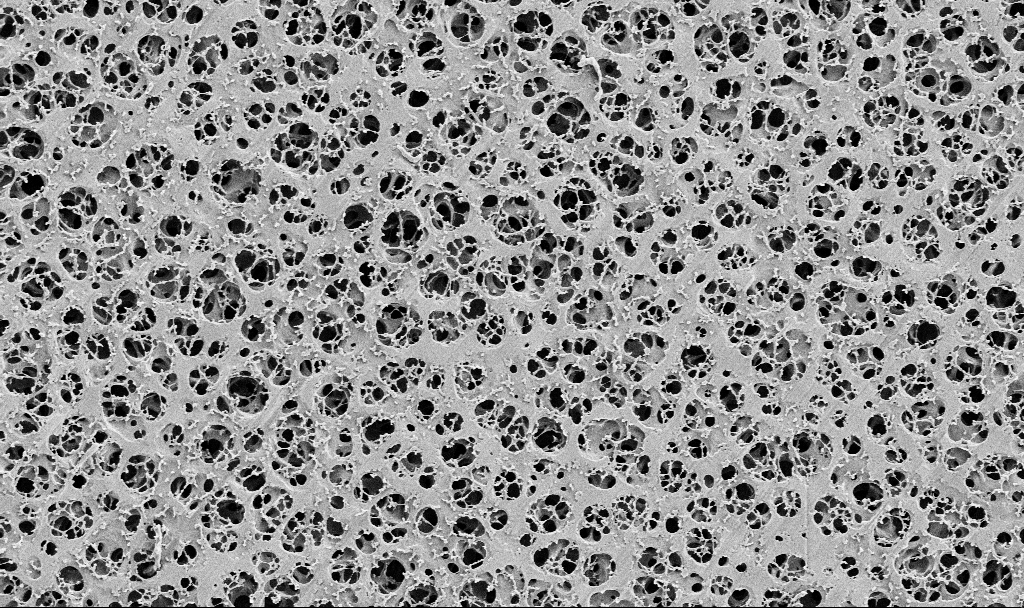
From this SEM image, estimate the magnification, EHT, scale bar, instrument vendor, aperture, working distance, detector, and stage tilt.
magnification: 5 K X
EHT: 2 kV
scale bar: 10000 nm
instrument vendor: Zeiss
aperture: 30 µm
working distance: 3.7 mm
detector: SE2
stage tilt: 0°